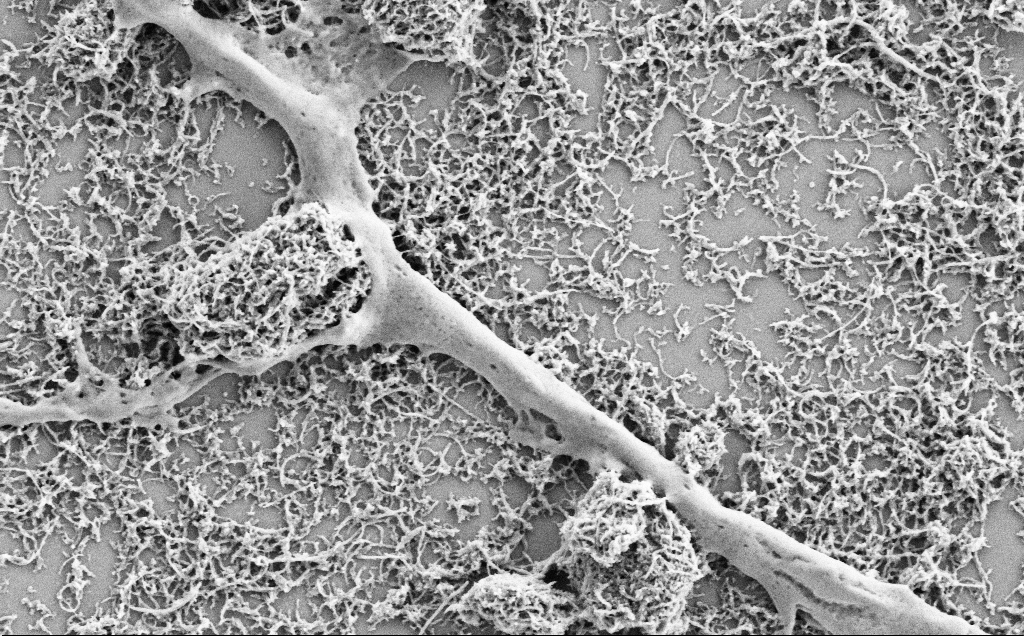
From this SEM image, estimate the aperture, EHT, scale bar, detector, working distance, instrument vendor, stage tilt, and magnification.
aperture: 30 µm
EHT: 2 kV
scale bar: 1000 nm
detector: SE2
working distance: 7.1 mm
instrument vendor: Zeiss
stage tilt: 0°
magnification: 25 K X